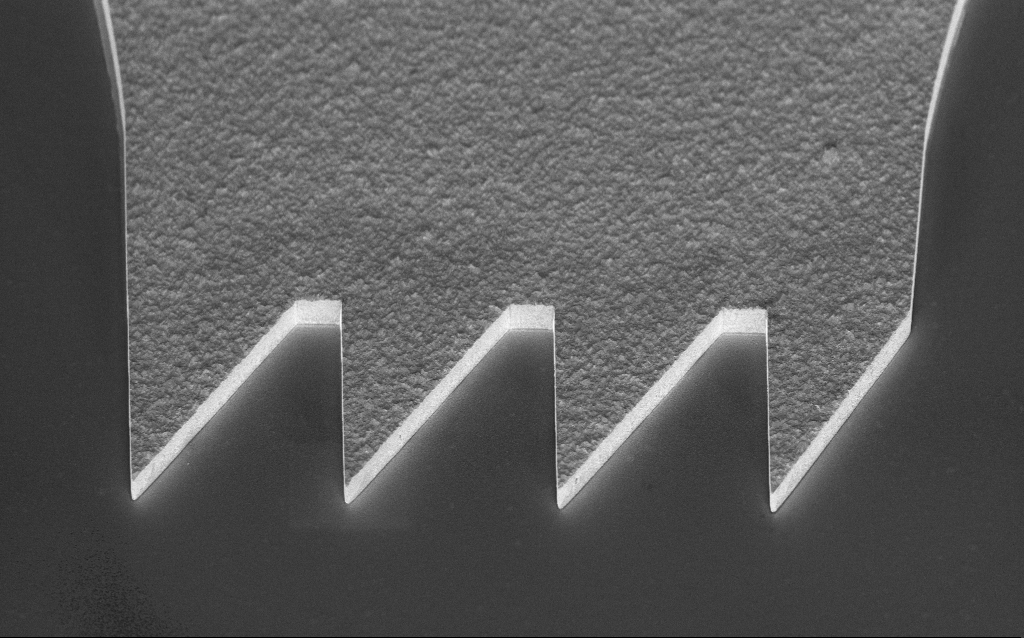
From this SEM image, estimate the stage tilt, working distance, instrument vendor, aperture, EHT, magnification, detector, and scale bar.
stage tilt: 45°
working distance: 6.4 mm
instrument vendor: Zeiss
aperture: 30 µm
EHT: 3 kV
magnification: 6.9 K X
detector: InLens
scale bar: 10000 nm